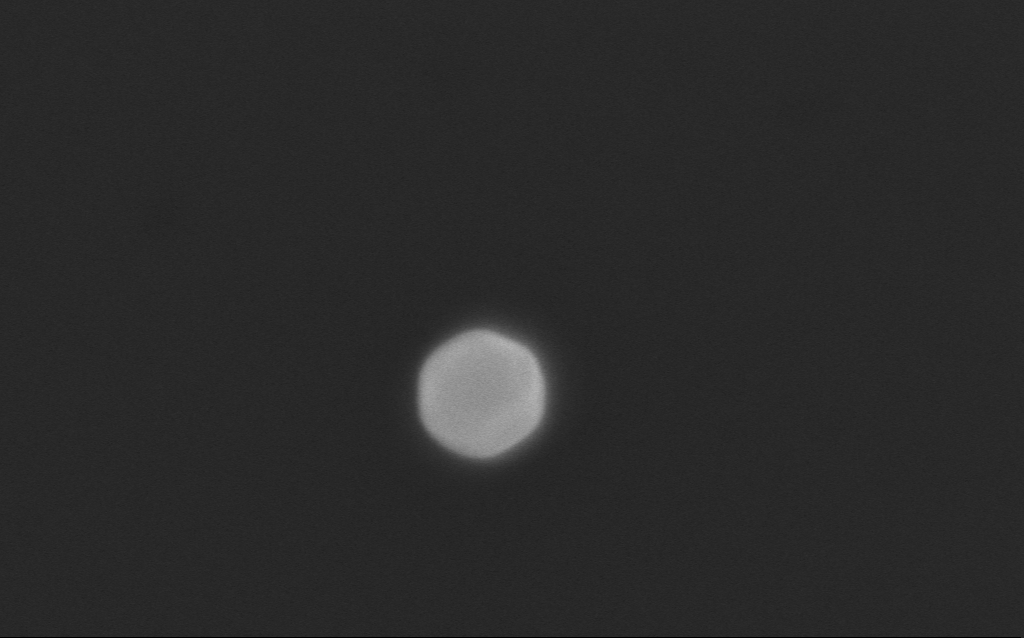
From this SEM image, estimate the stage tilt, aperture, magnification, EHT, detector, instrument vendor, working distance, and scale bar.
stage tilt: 0°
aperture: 30 µm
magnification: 502 K X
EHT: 10 kV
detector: InLens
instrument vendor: Zeiss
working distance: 4 mm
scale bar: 100 nm